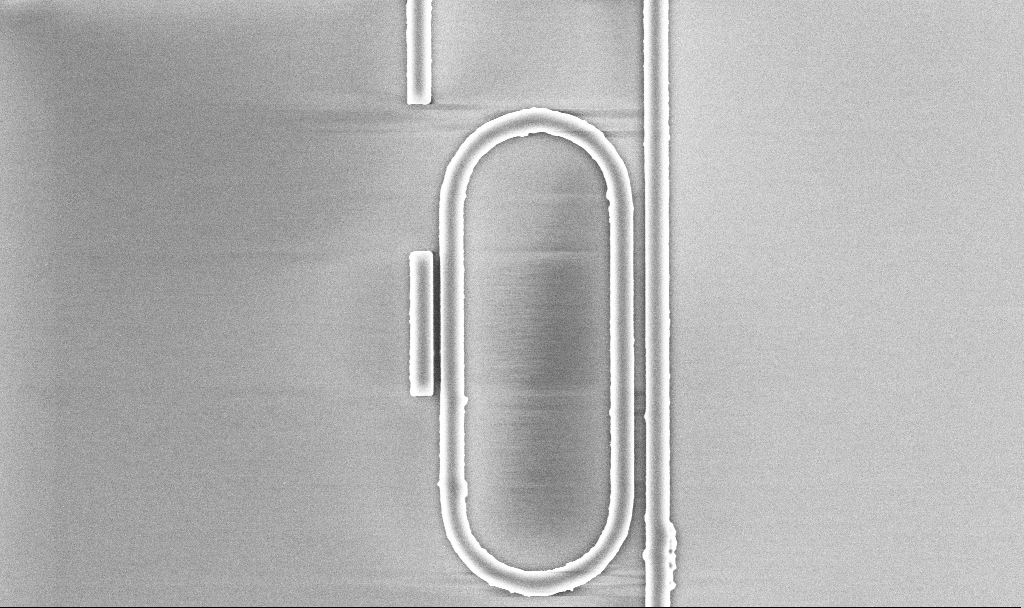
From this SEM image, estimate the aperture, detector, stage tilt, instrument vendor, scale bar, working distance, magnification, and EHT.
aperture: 30 µm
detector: InLens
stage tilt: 0°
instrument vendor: Zeiss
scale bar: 2000 nm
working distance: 5.2 mm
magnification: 18.11 K X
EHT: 5 kV